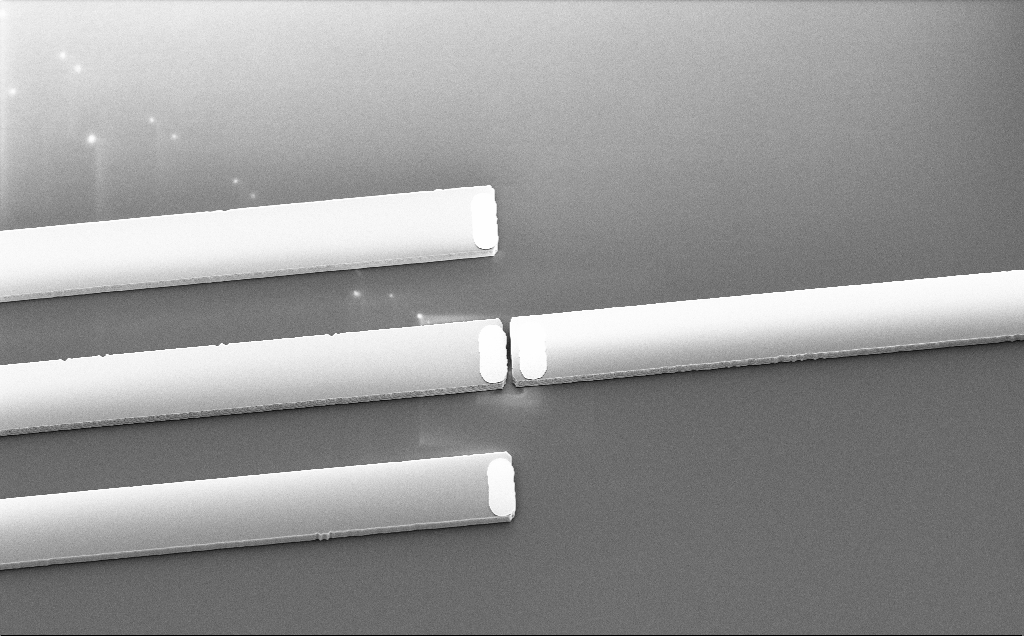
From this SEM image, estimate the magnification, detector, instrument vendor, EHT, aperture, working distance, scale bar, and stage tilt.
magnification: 1.07 K X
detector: SE2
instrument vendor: Zeiss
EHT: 5 kV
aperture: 30 µm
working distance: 9 mm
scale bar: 20000 nm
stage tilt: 34.3°